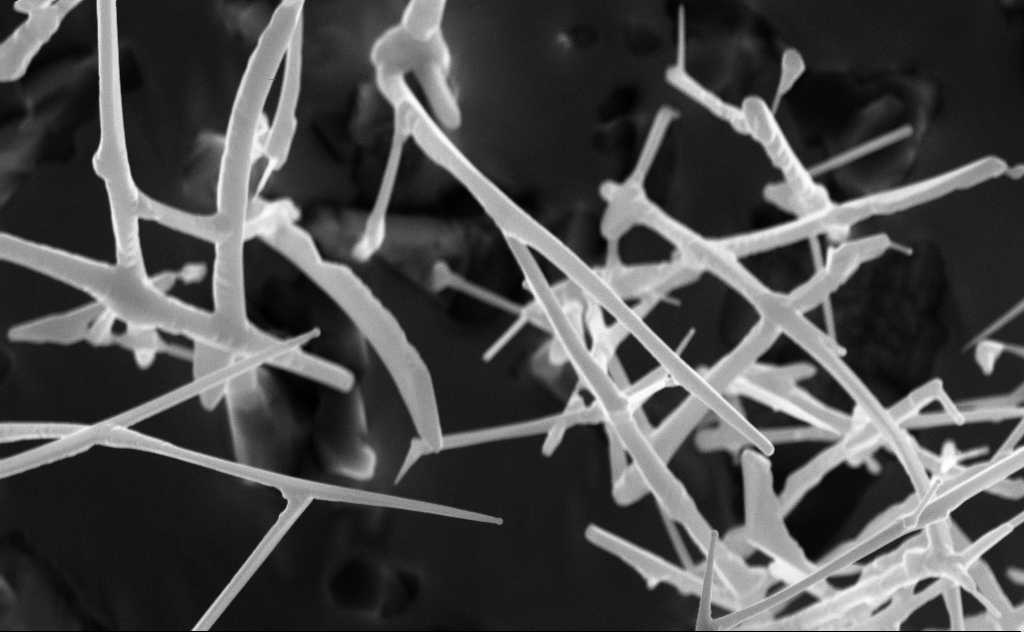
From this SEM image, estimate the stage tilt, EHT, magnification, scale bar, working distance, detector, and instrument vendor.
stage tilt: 0°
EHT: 10 kV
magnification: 40 K X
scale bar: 1000 nm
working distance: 6 mm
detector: InLens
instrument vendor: Zeiss